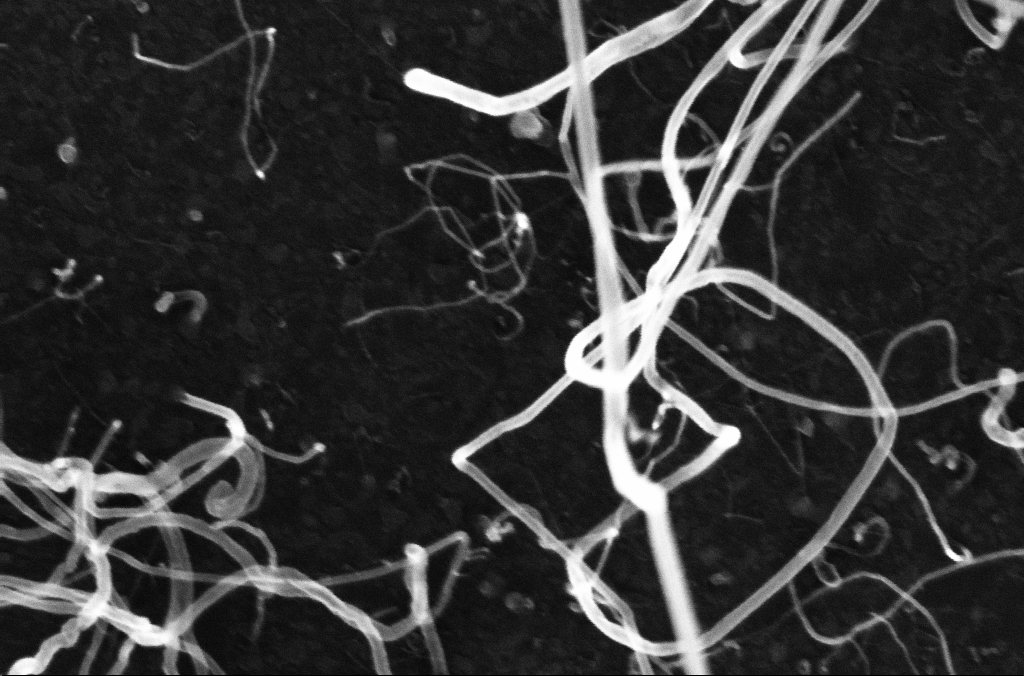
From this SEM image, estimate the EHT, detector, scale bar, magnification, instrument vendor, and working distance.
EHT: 10 kV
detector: InLens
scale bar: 100 nm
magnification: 200 K X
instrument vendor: Zeiss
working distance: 3.3 mm